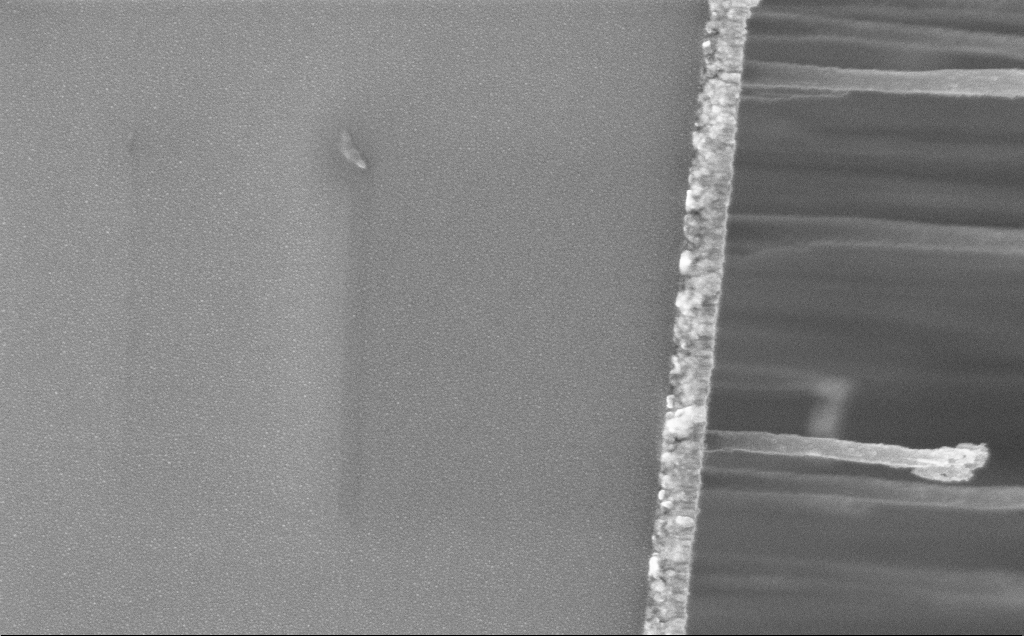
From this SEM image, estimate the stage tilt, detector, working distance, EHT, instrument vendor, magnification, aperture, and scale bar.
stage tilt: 0°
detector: InLens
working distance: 12 mm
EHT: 5 kV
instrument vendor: Zeiss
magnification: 62.46 K X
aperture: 30 µm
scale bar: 1000 nm